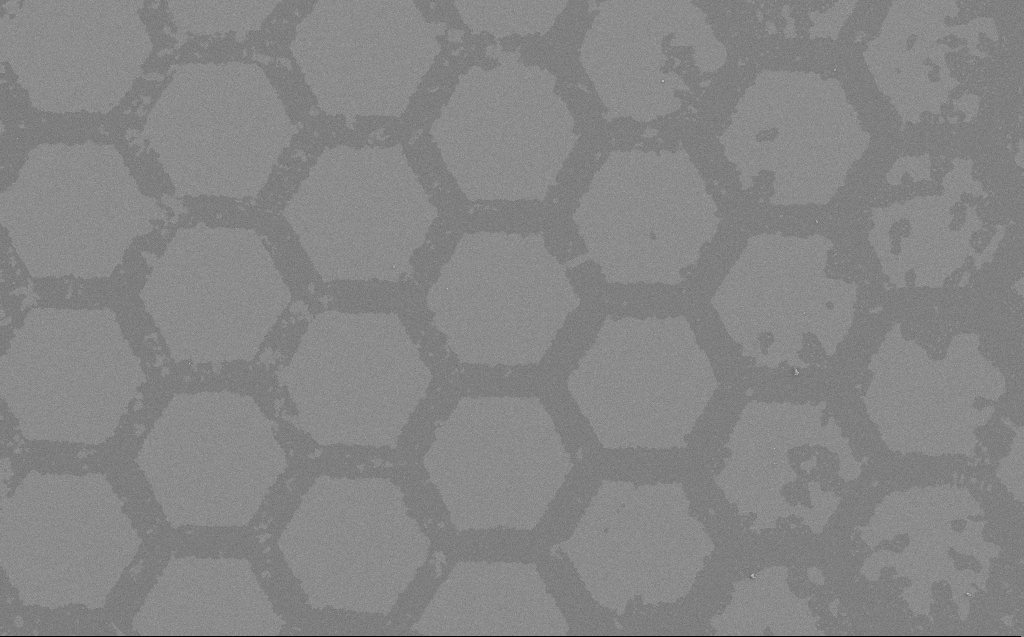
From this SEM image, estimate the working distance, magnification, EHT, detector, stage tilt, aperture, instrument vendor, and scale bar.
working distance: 5 mm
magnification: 1.02 K X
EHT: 3 kV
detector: SE2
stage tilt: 0°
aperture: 30 µm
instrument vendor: Zeiss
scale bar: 20000 nm